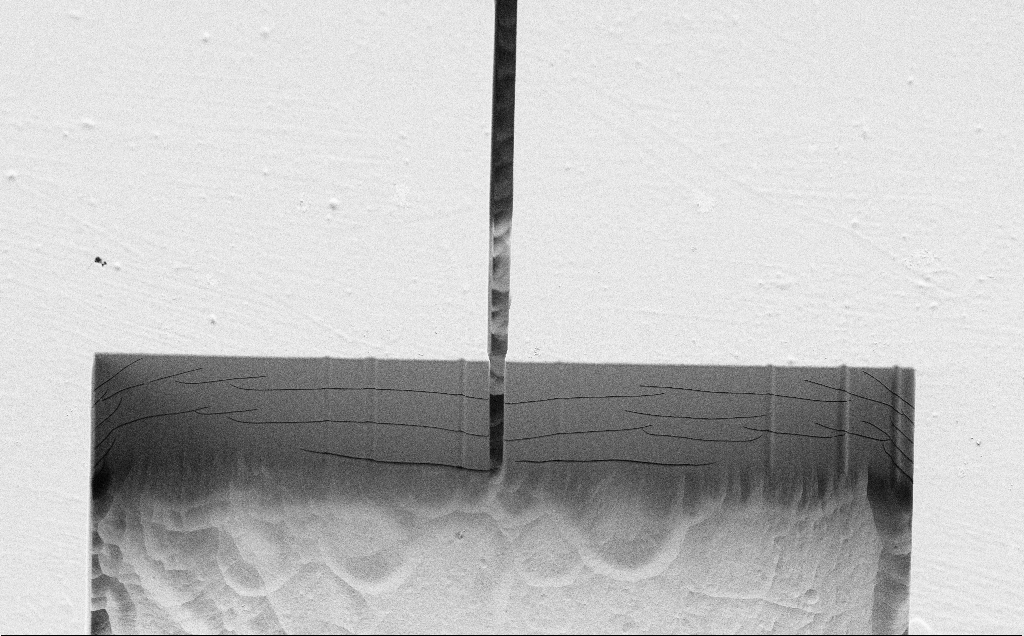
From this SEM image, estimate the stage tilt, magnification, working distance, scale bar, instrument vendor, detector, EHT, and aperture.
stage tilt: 45°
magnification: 0.756 K X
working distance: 5 mm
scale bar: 20000 nm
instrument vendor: Zeiss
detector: SE2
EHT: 1.2 kV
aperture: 30 µm